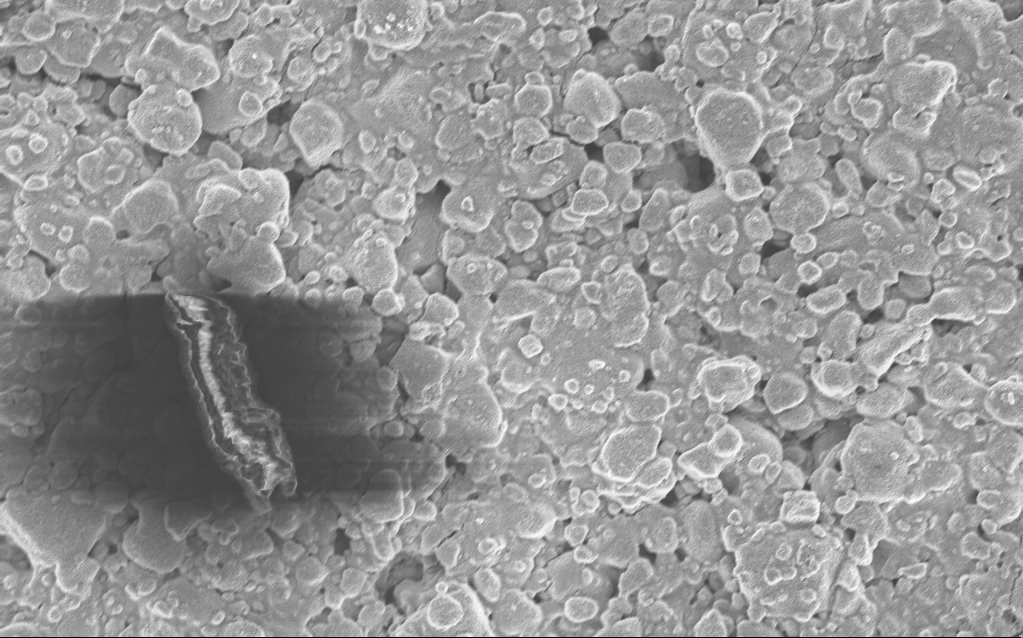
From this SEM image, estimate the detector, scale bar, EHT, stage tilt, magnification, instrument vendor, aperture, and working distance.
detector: InLens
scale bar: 10000 nm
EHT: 5 kV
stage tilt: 0°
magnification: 3.88 K X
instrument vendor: Zeiss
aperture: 30 µm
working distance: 4.8 mm